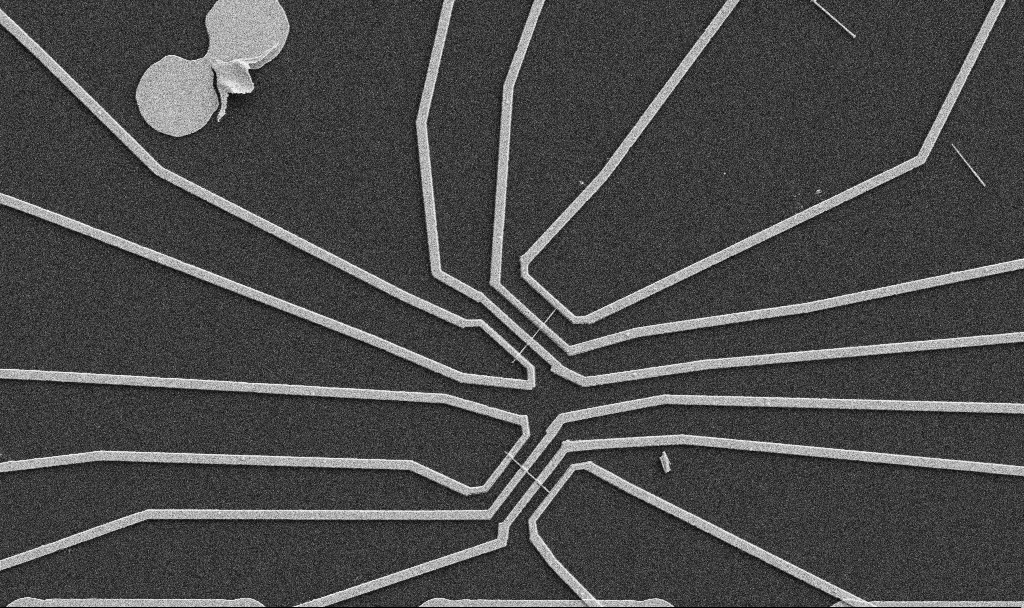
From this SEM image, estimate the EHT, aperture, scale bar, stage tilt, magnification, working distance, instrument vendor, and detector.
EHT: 5 kV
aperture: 30 µm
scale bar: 10000 nm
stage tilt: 0°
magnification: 5 K X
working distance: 10.7 mm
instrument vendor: Zeiss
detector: SE2